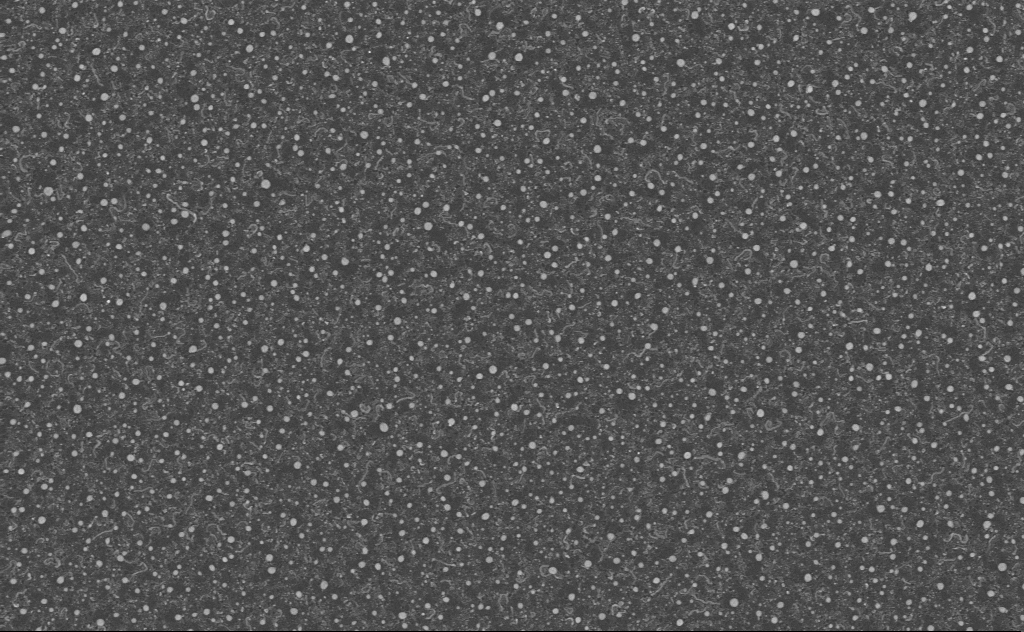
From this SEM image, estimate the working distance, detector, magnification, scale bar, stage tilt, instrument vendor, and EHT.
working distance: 4 mm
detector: InLens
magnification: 40 K X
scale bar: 1000 nm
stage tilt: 0°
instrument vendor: Zeiss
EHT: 3 kV